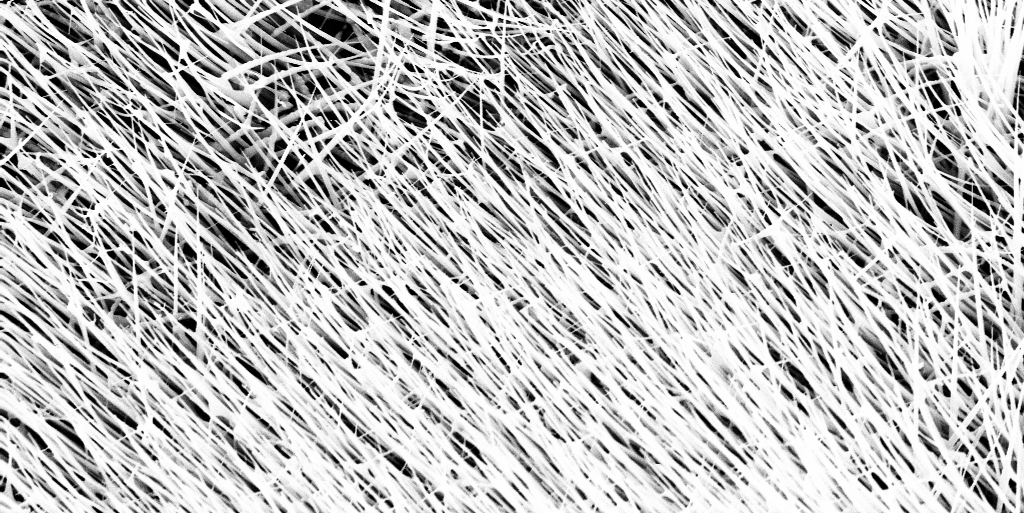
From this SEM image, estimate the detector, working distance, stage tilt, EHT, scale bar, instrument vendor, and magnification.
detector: InLens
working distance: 13 mm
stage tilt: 0°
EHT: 10 kV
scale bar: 2000 nm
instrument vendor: Zeiss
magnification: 20 K X